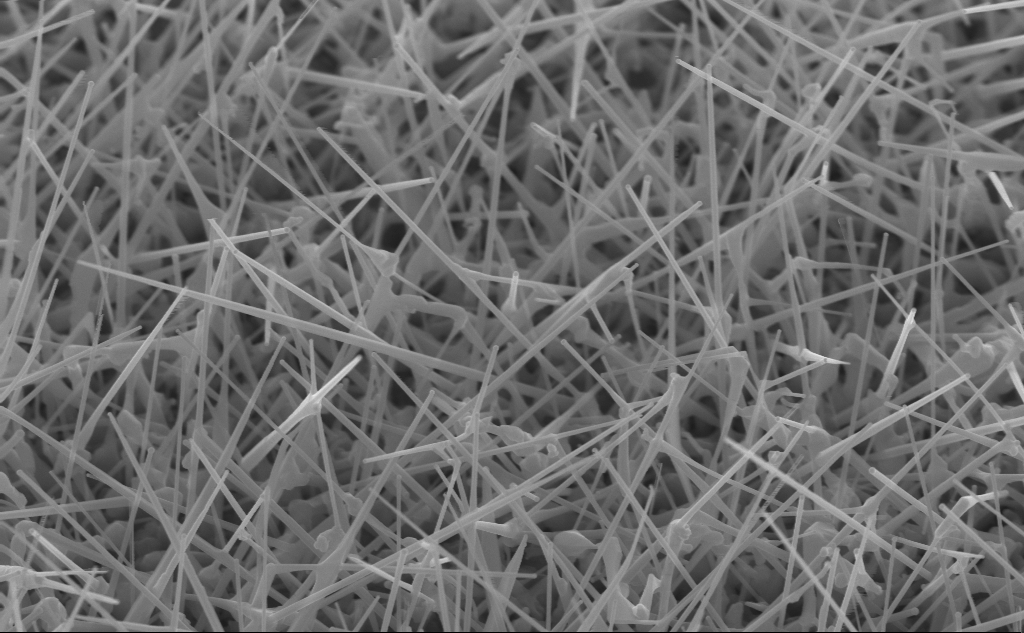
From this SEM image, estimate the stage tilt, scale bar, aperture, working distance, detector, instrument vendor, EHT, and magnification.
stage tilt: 45°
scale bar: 1000 nm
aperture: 30 µm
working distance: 5 mm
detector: InLens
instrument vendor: Zeiss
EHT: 10 kV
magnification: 46.97 K X